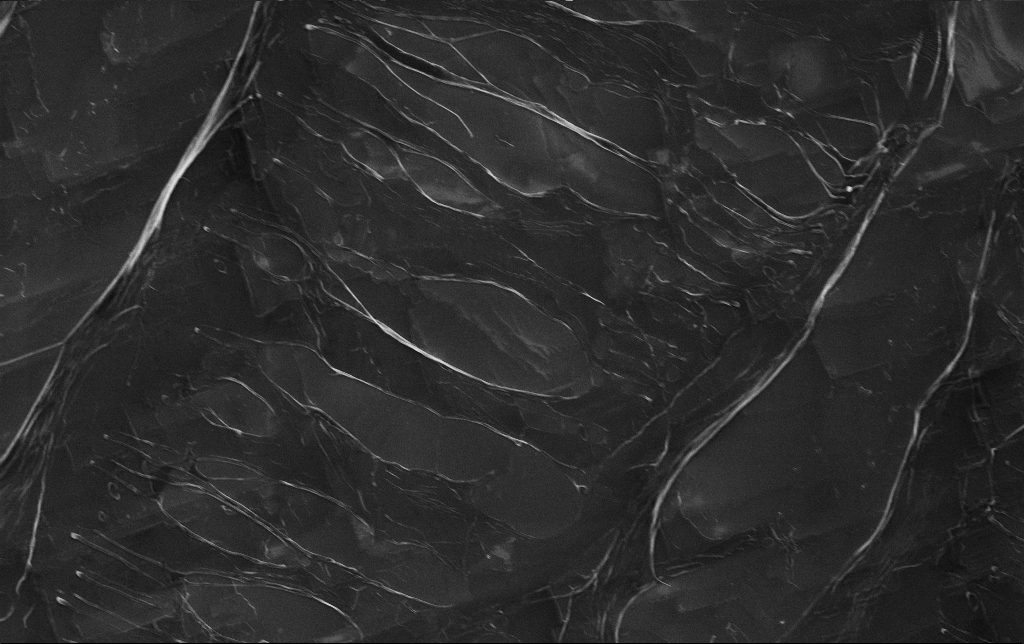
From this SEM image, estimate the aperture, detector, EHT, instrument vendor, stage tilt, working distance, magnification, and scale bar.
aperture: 30 µm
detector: InLens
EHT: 5 kV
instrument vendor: Zeiss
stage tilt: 0°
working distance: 3.1 mm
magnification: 3.94 K X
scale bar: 10000 nm